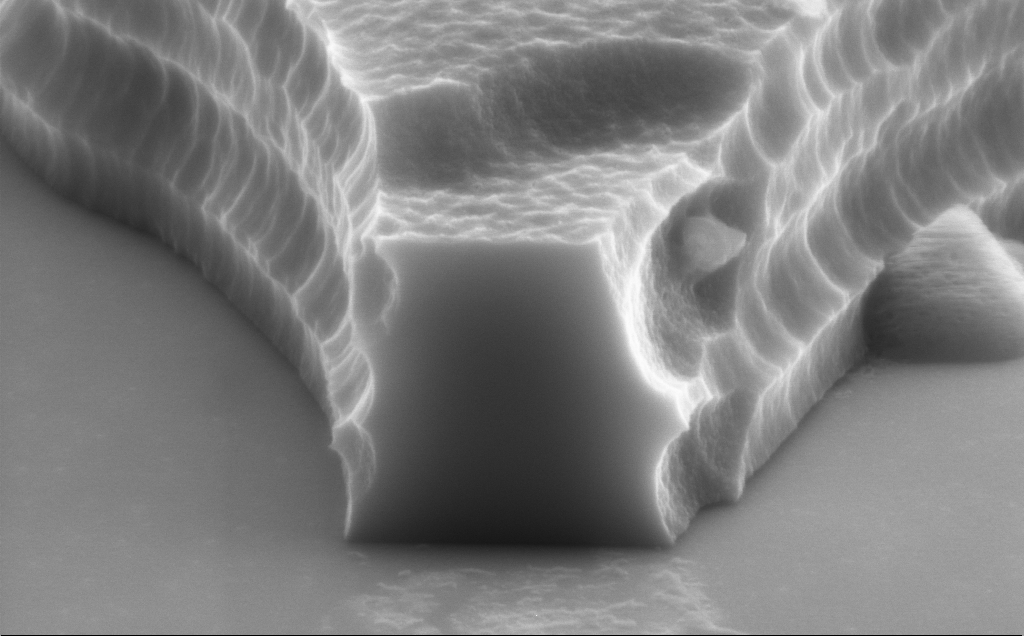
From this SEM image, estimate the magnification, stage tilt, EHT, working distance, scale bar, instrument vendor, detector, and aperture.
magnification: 70.2 K X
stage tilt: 70°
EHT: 8 kV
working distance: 12 mm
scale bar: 1000 nm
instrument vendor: Zeiss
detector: SE2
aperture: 30 µm